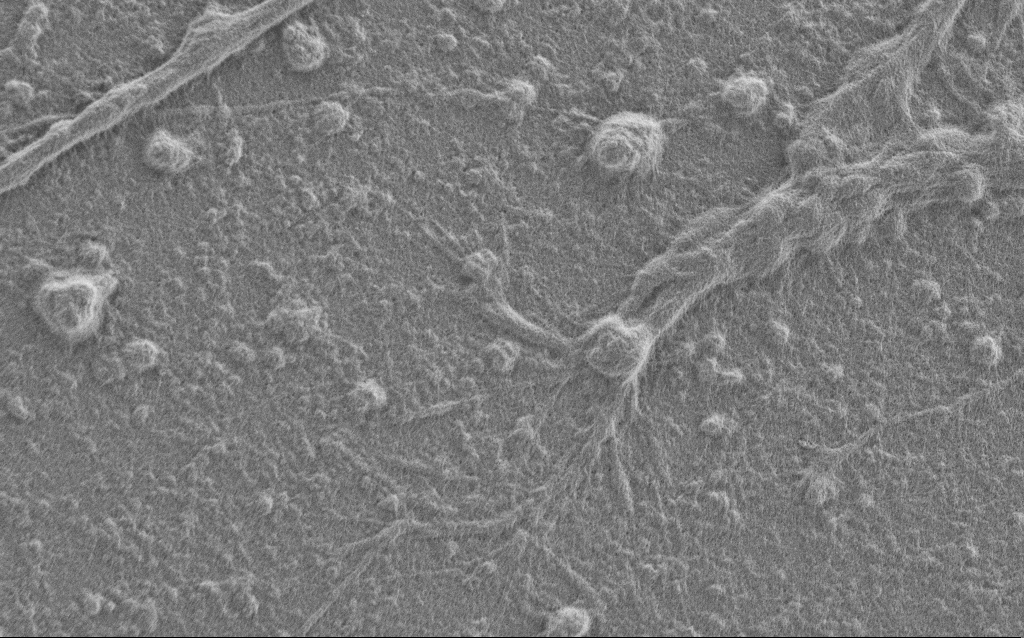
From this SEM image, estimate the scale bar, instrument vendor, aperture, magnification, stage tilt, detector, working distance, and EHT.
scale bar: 2000 nm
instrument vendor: Zeiss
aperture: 30 µm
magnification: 7.5 K X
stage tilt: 0°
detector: SE2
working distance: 6 mm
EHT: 1 kV